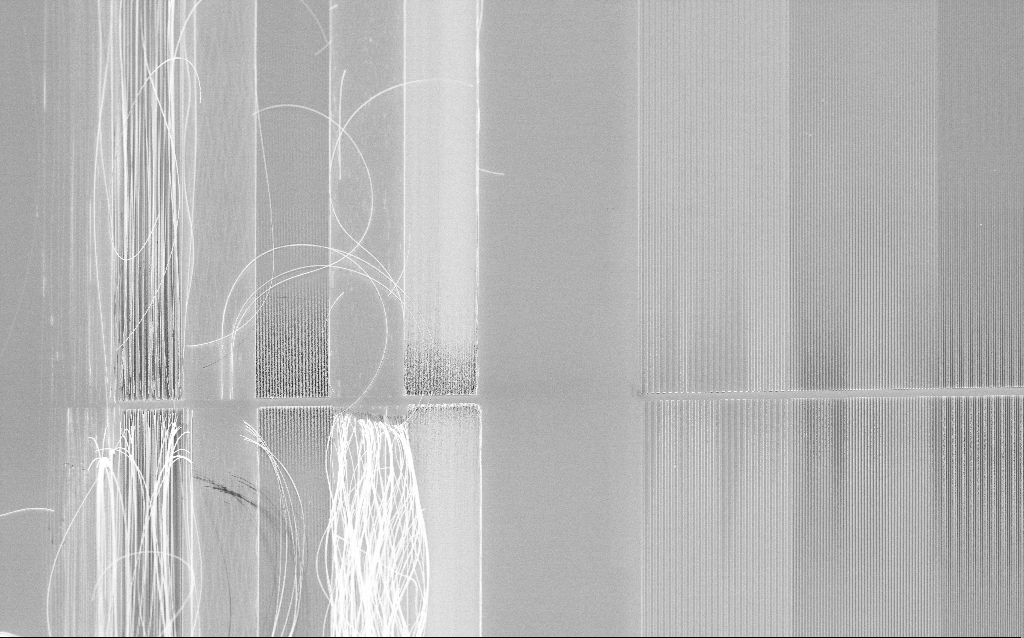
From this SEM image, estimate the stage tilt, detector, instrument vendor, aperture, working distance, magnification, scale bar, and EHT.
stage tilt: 30°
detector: InLens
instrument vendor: Zeiss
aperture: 30 µm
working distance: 4.1 mm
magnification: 1.1 K X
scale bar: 20000 nm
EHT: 5 kV